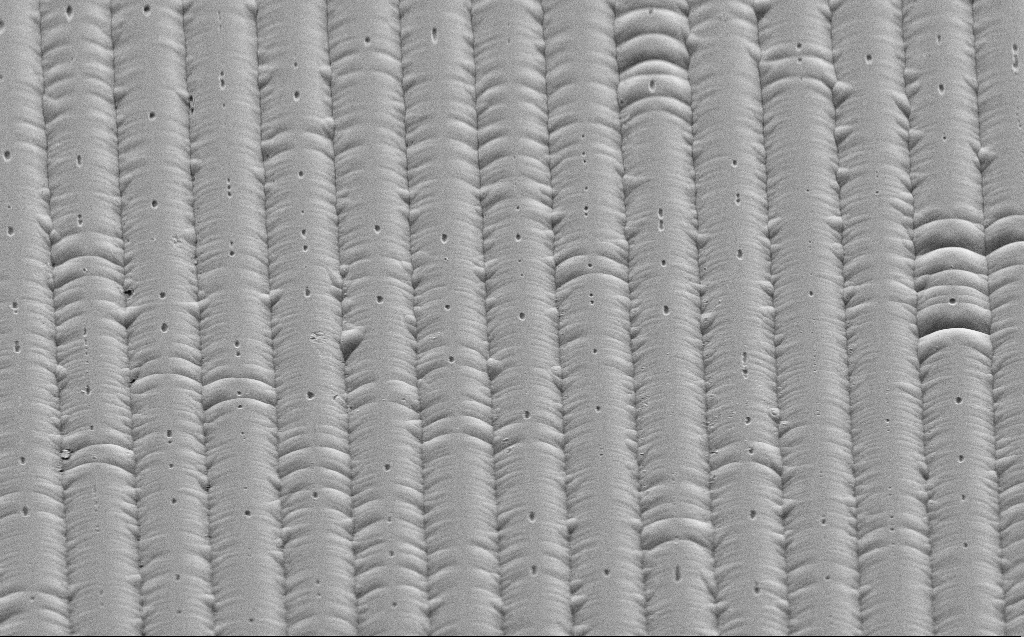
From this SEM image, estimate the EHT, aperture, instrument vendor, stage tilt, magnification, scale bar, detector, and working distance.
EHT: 3 kV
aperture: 30 µm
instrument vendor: Zeiss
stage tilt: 45°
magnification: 2.66 K X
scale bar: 10000 nm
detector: SE2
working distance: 6 mm